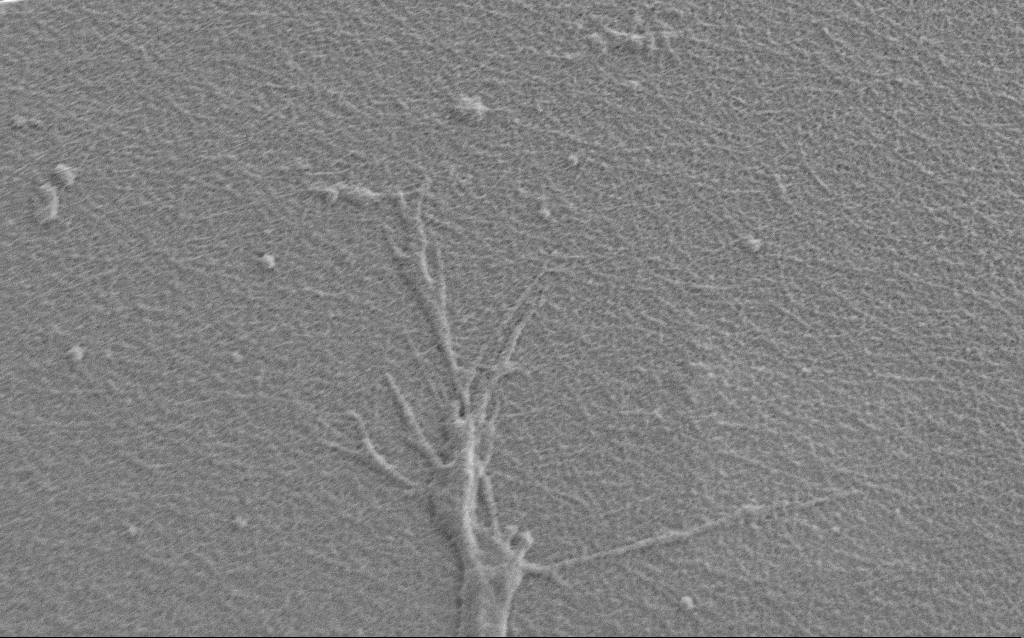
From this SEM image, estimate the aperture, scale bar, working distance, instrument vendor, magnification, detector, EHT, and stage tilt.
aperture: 30 µm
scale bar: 2000 nm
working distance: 7 mm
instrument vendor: Zeiss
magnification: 10 K X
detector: SE2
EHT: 0.9 kV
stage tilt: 0°